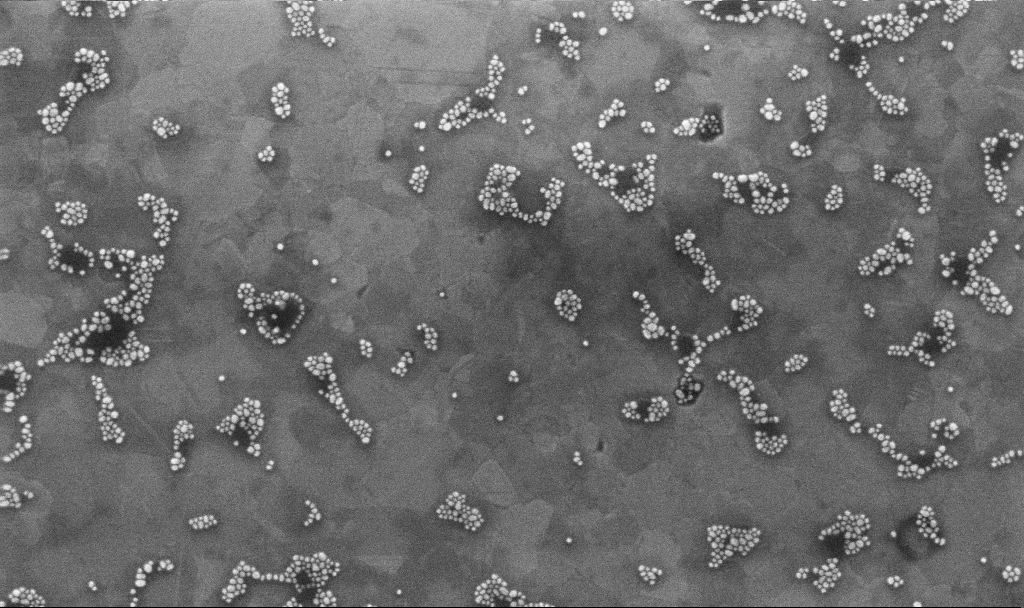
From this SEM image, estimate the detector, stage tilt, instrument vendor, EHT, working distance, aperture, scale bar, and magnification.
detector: InLens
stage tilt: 0°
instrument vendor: Zeiss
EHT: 10 kV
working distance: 3.1 mm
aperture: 30 µm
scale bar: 200 nm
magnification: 100.18 K X